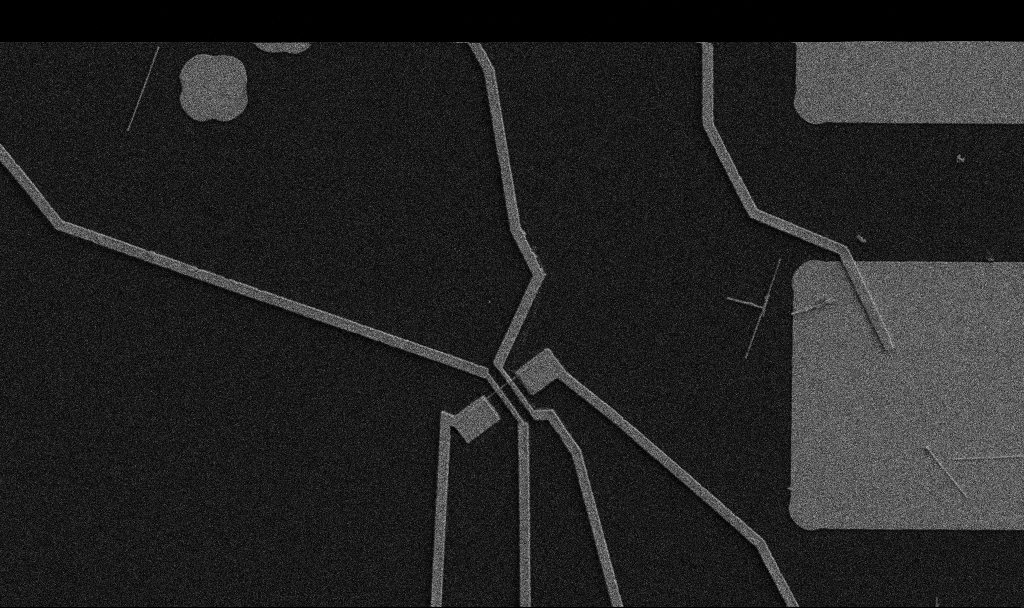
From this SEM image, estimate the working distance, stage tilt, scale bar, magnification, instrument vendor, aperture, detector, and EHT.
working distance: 10.7 mm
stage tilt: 0°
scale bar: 10000 nm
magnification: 5 K X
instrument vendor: Zeiss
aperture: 30 µm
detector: SE2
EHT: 5 kV